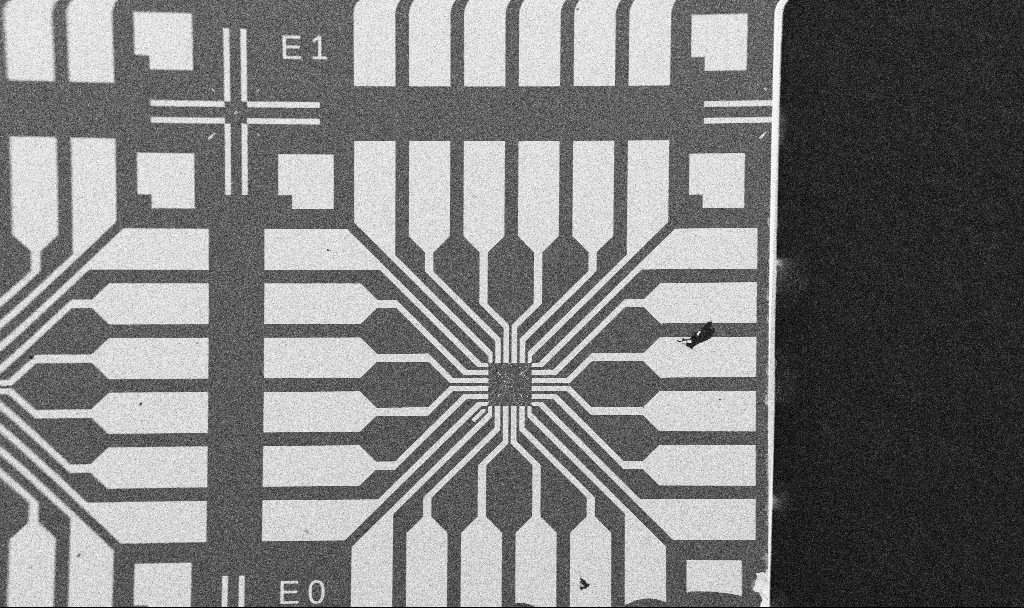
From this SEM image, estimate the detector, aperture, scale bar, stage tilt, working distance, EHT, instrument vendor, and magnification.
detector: SE2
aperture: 30 µm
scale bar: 200000 nm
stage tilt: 0°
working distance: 10.7 mm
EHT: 5 kV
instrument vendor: Zeiss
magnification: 0.1 K X